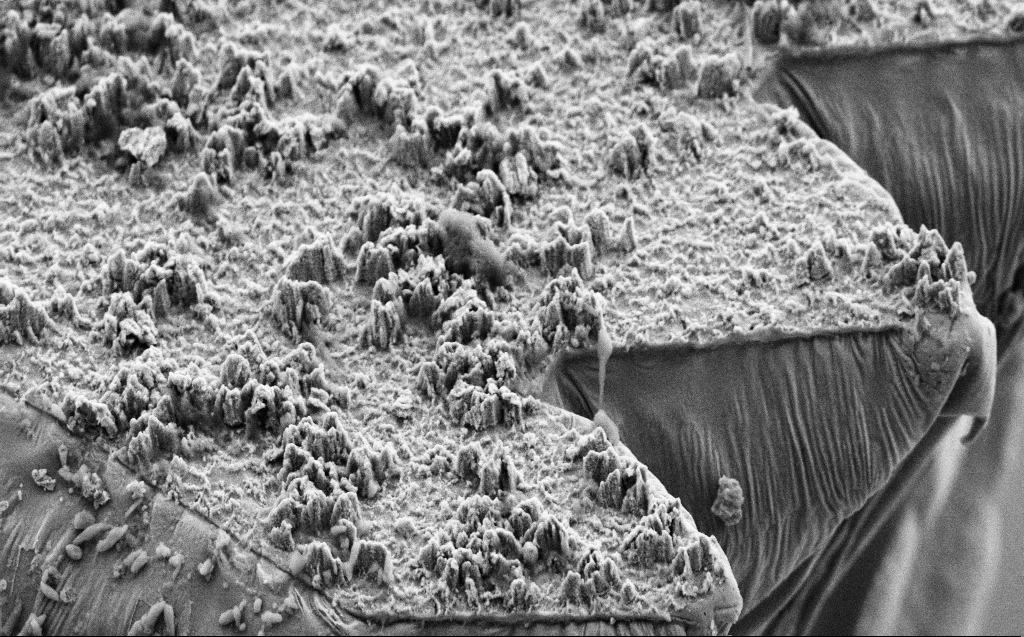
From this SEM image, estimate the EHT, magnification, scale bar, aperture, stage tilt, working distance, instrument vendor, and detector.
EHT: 5 kV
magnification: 12.21 K X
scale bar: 1000 nm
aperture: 30 µm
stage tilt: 45°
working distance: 9 mm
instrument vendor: Zeiss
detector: SE2